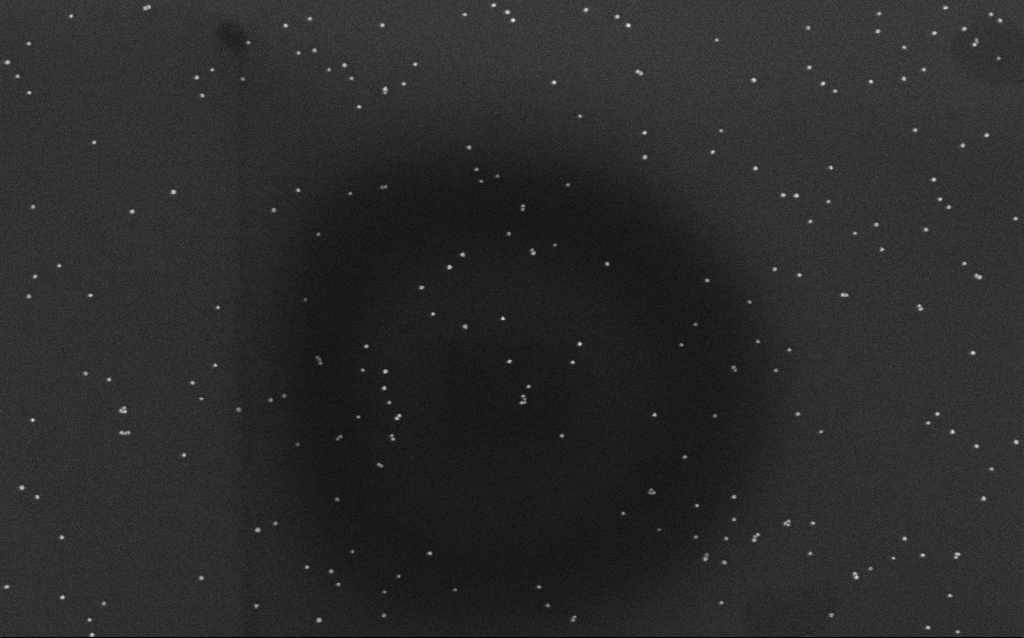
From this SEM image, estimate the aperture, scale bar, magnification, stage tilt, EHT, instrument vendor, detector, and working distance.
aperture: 30 µm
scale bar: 200 nm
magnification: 100 K X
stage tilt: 0°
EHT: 10 kV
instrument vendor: Zeiss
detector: InLens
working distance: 6.6 mm